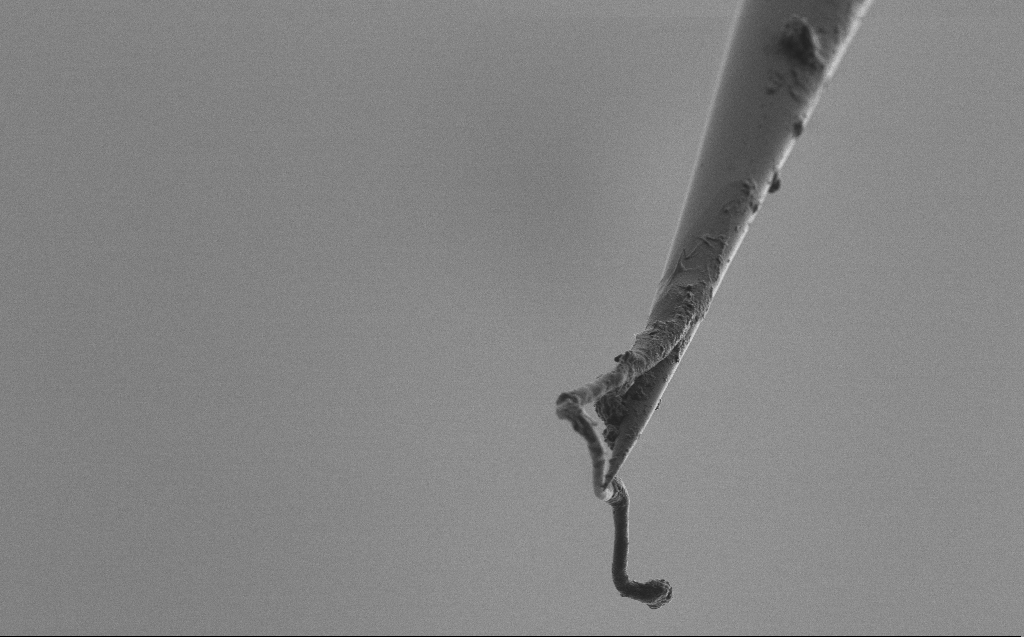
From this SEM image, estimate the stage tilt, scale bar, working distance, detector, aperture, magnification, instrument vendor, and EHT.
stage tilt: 45°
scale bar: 10000 nm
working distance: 3 mm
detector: SE2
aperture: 30 µm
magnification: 5 K X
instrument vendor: Zeiss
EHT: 1 kV